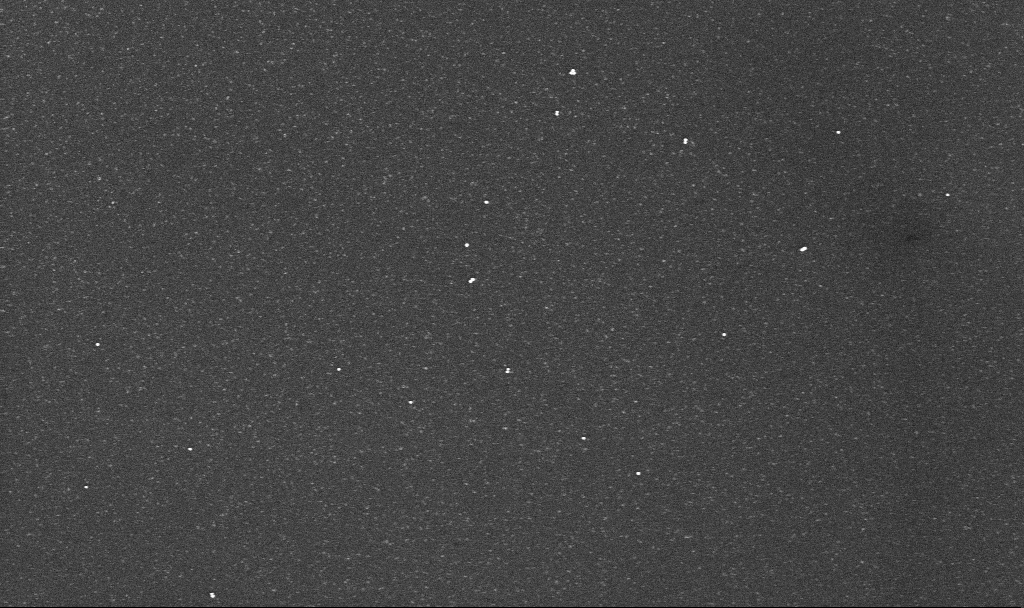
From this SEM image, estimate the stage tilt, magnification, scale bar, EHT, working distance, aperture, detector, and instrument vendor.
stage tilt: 0°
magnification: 43.83 K X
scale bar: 1000 nm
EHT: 10 kV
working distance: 3.1 mm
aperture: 30 µm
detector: InLens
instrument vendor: Zeiss